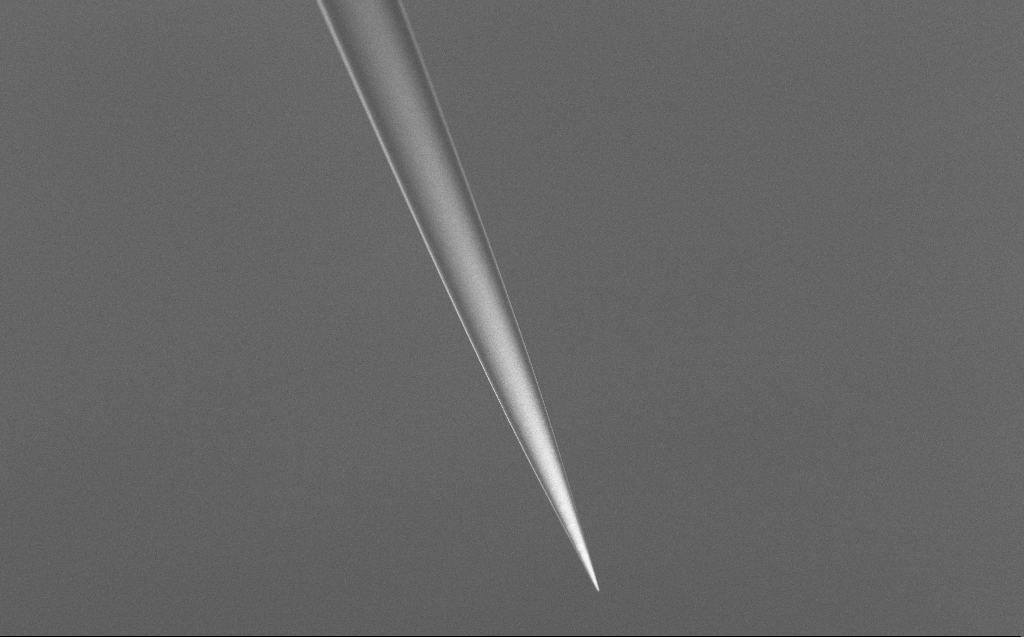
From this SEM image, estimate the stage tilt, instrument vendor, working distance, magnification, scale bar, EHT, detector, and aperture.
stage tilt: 45°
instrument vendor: Zeiss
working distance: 6 mm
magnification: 1 K X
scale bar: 20000 nm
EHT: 5 kV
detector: InLens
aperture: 30 µm